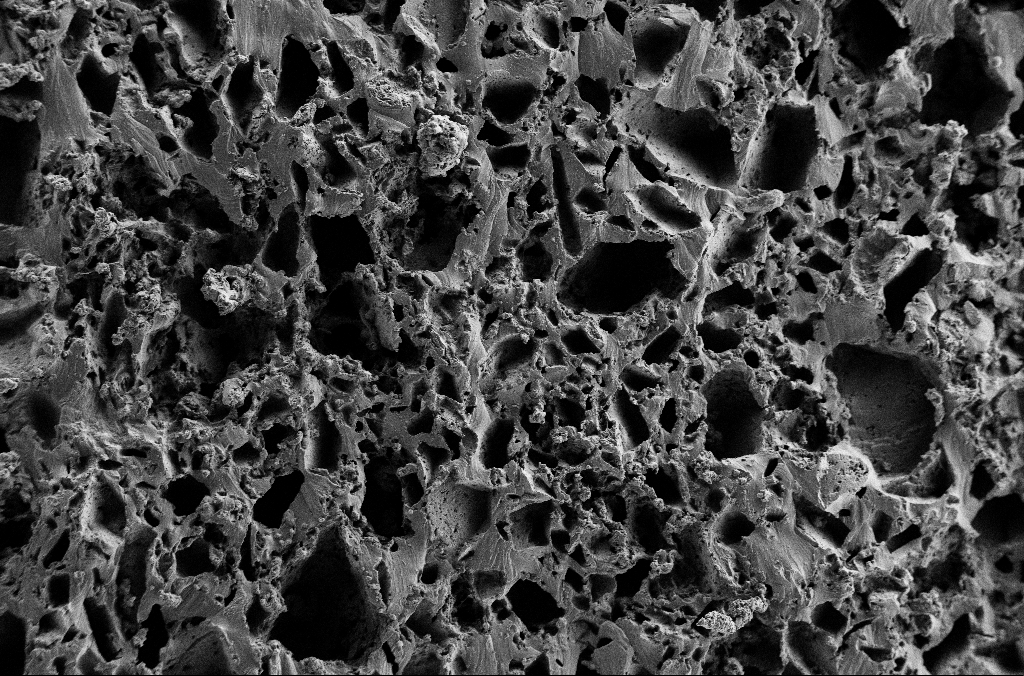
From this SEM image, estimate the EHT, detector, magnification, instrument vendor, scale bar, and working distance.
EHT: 2 kV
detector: SE2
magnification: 0.25 K X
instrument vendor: Zeiss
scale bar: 100000 nm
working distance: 3 mm